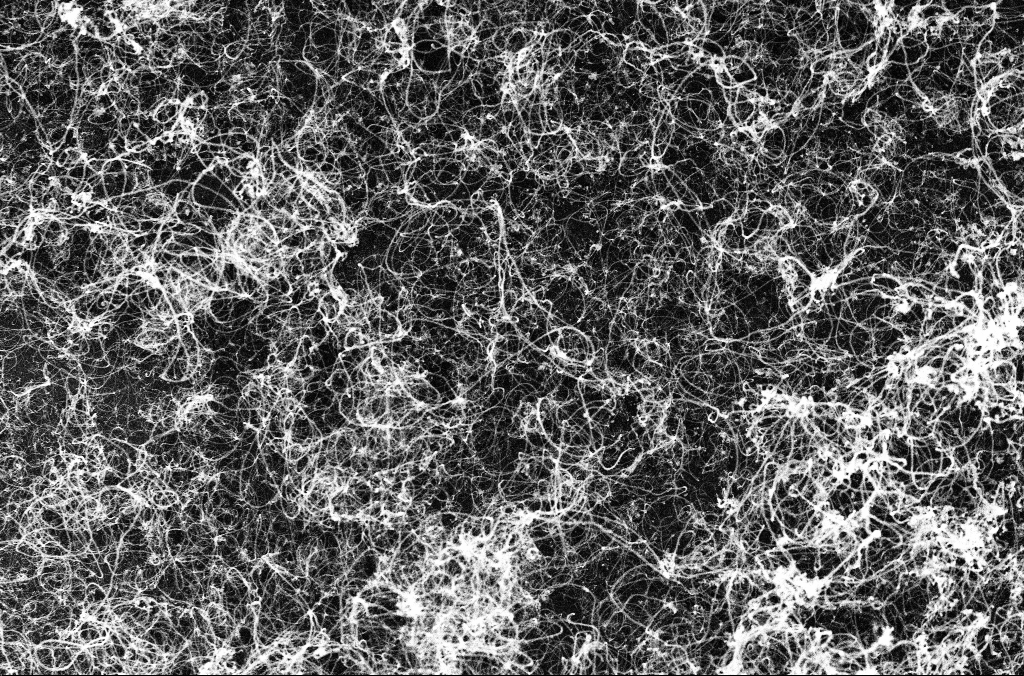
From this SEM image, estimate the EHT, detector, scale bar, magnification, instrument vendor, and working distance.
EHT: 10 kV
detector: InLens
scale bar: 2000 nm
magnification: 10 K X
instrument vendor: Zeiss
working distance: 3.3 mm